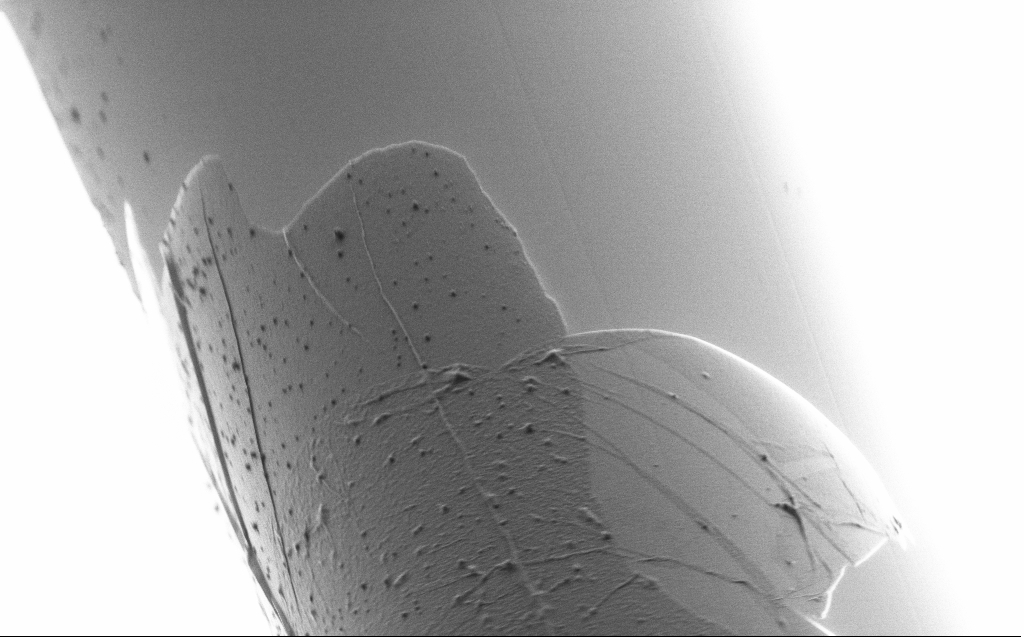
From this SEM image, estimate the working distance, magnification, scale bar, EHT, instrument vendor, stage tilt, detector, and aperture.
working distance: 4 mm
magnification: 10 K X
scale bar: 2000 nm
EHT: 1 kV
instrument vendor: Zeiss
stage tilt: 45°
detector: SE2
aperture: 30 µm